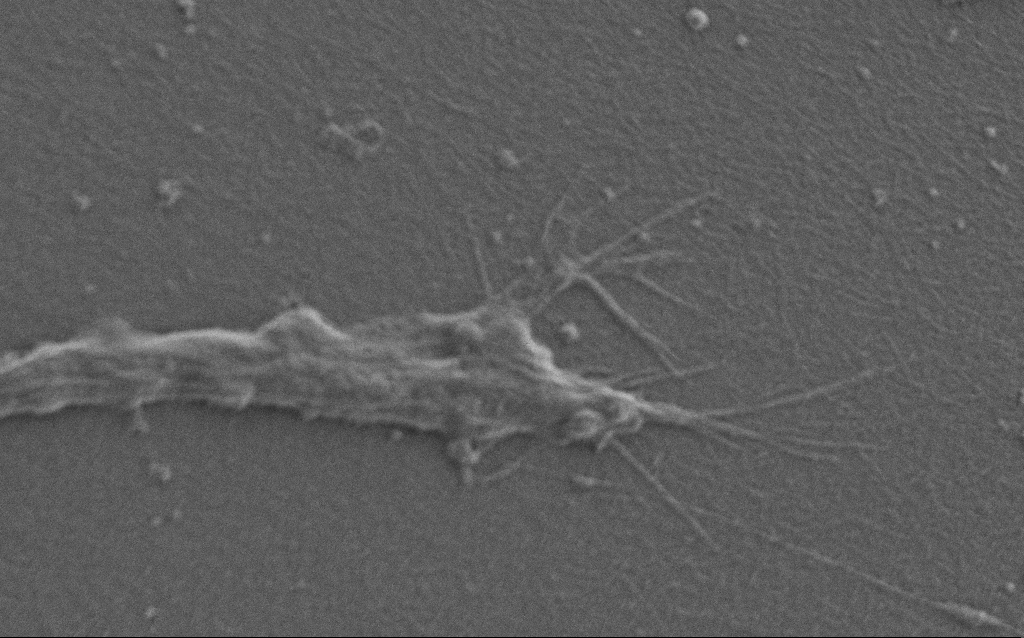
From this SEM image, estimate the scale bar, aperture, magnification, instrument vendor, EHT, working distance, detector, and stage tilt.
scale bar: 2000 nm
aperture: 30 µm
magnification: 10 K X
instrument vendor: Zeiss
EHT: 0.9 kV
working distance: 7 mm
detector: SE2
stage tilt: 0°